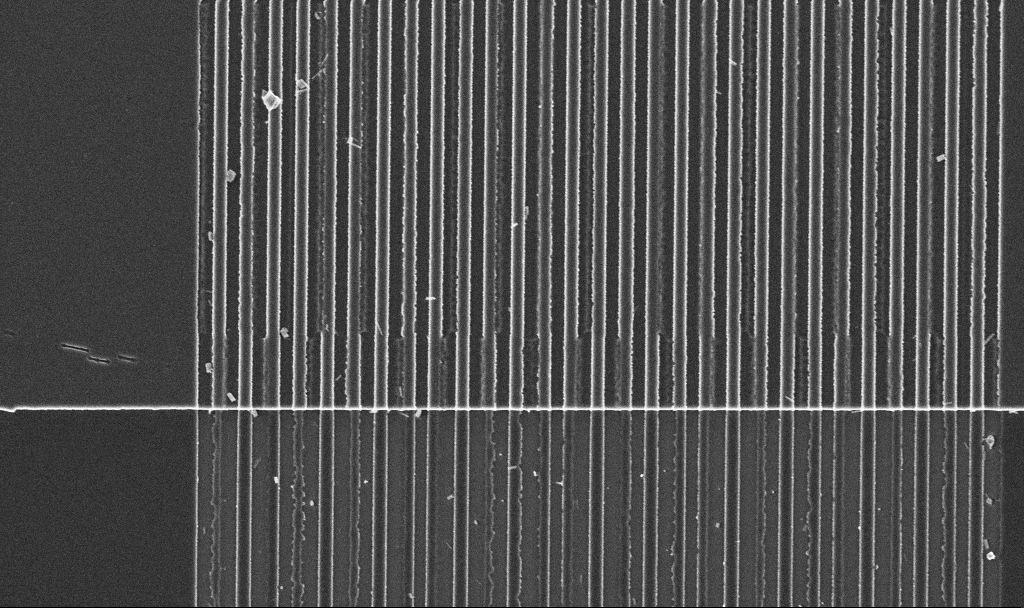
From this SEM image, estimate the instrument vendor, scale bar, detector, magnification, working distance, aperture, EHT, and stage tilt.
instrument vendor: Zeiss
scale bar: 2000 nm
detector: InLens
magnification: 15.23 K X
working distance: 2.9 mm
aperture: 30 µm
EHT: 3 kV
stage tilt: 0°